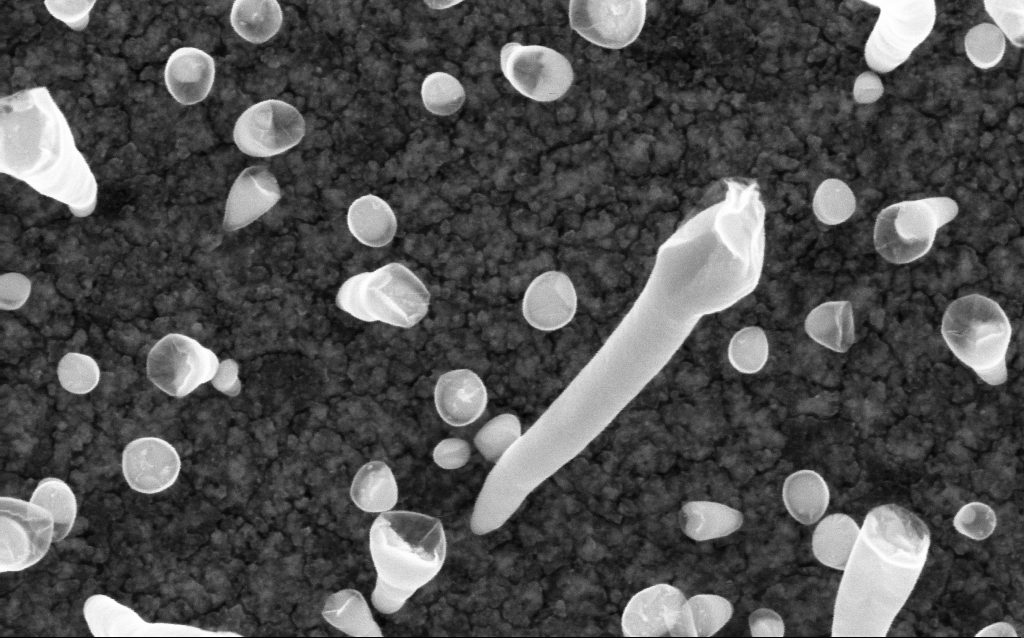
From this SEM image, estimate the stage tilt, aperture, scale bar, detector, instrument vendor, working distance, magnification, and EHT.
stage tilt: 0°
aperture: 30 µm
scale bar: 100 nm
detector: InLens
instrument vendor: Zeiss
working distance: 2 mm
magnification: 200 K X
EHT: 5 kV